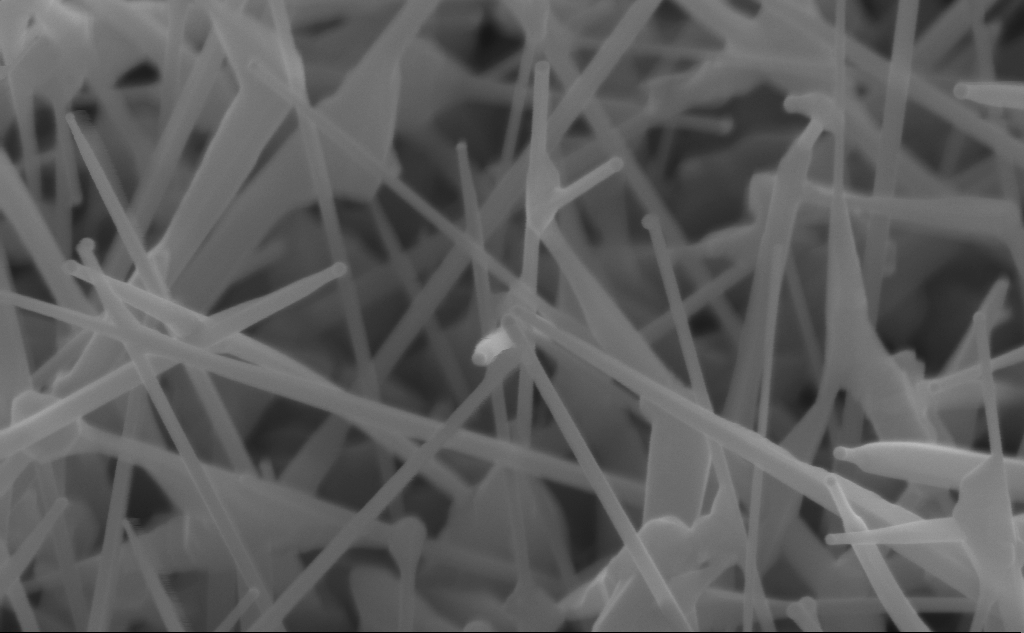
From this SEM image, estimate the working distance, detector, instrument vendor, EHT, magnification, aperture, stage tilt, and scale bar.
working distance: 4 mm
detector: InLens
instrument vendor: Zeiss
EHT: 10 kV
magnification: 150 K X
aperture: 30 µm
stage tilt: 45°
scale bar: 200 nm